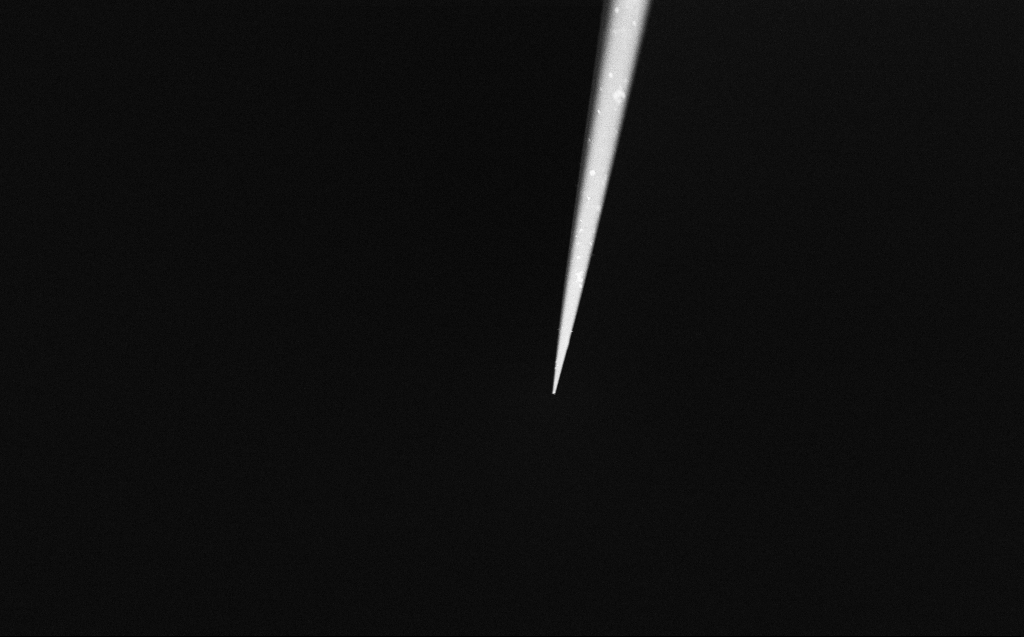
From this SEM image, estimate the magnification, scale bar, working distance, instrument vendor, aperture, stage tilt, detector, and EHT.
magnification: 5 K X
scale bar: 10000 nm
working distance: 4 mm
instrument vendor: Zeiss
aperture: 30 µm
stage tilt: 45°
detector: InLens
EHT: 2 kV